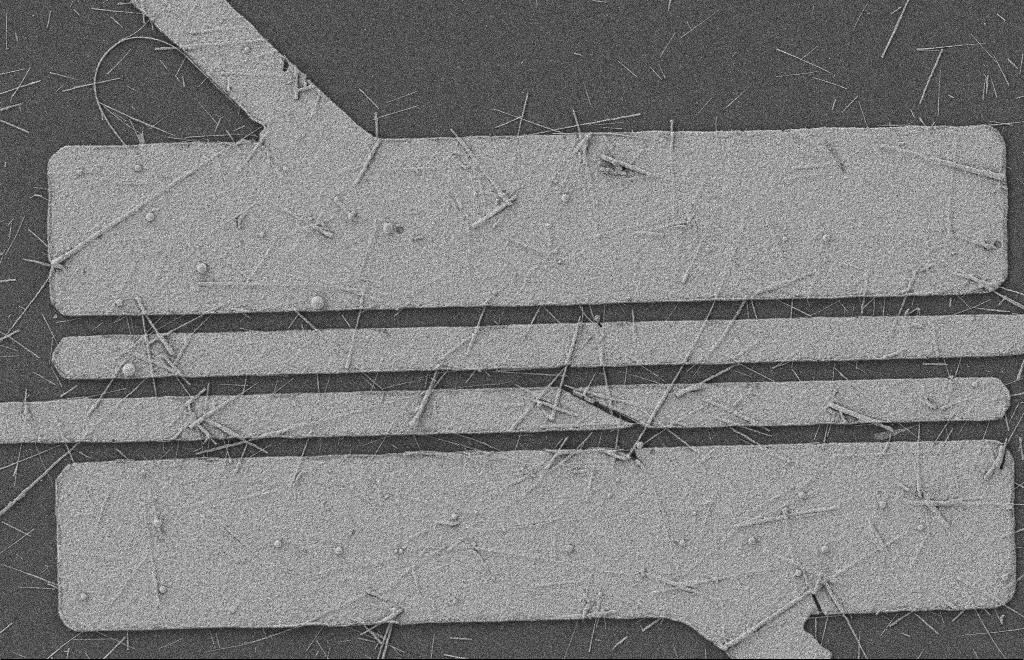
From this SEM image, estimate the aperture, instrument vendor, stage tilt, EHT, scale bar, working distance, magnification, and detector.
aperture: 20 µm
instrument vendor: Zeiss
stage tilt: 0°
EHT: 2 kV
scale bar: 2000 nm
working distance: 8 mm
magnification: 5.77 K X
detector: SE2